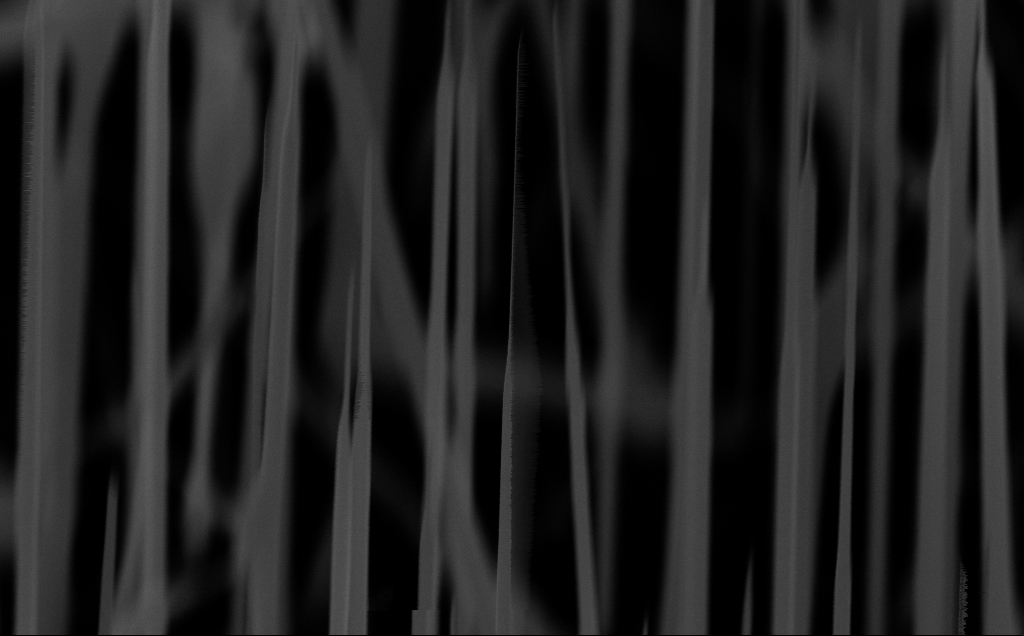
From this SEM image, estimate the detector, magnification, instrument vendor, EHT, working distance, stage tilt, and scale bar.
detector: InLens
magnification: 79.81 K X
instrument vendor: Zeiss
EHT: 10 kV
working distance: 6 mm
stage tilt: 45°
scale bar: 200 nm